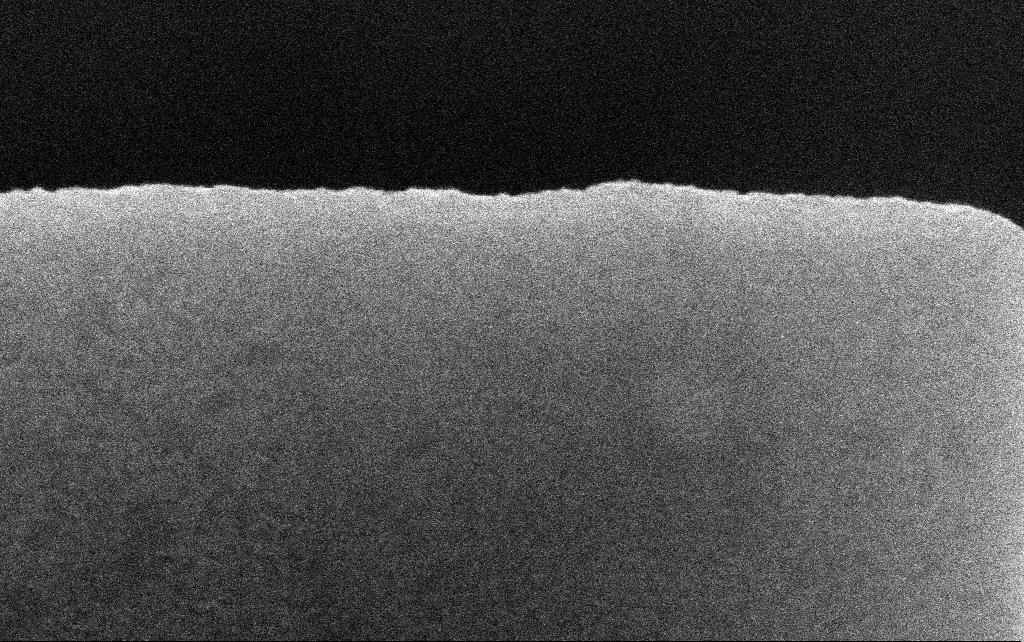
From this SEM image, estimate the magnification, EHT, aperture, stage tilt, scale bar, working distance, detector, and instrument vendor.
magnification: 262.28 K X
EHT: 10 kV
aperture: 30 µm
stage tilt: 0°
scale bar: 200 nm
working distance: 2.5 mm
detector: InLens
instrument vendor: Zeiss